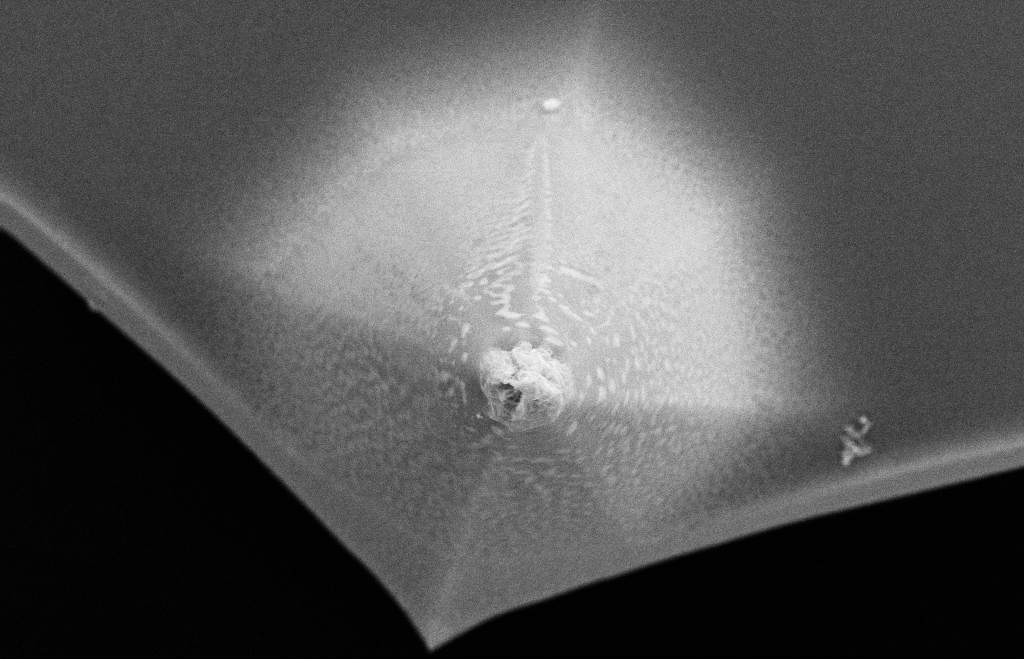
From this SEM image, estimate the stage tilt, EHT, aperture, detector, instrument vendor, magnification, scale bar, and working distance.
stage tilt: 0°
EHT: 10 kV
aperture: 30 µm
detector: SE2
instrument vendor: Zeiss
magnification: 15.45 K X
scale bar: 2000 nm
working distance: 8 mm